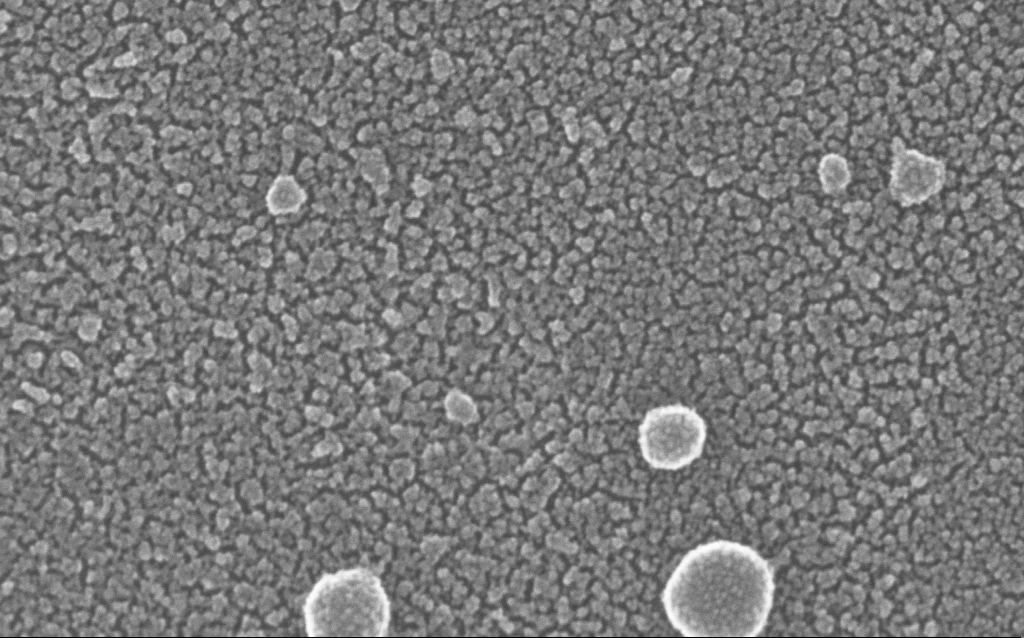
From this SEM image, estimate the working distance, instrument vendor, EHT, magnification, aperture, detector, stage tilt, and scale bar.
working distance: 1.5 mm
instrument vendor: Zeiss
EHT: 20 kV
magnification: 500 K X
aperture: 30 µm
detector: InLens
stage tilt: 0°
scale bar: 100 nm